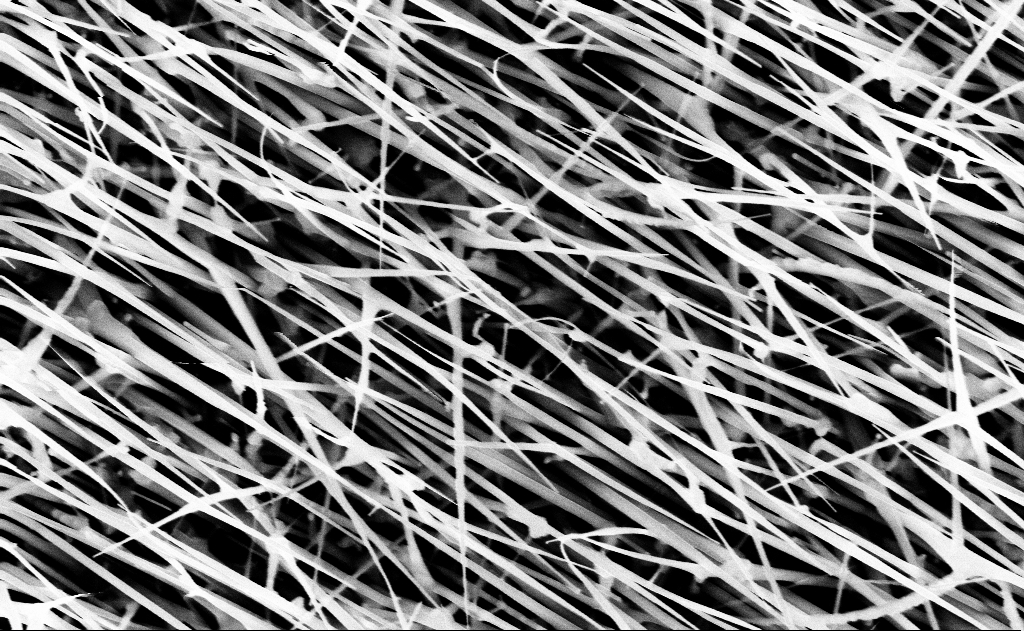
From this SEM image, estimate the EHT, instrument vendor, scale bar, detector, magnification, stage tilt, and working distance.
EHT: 10 kV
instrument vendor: Zeiss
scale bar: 1000 nm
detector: InLens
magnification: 40 K X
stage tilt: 0°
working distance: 13 mm